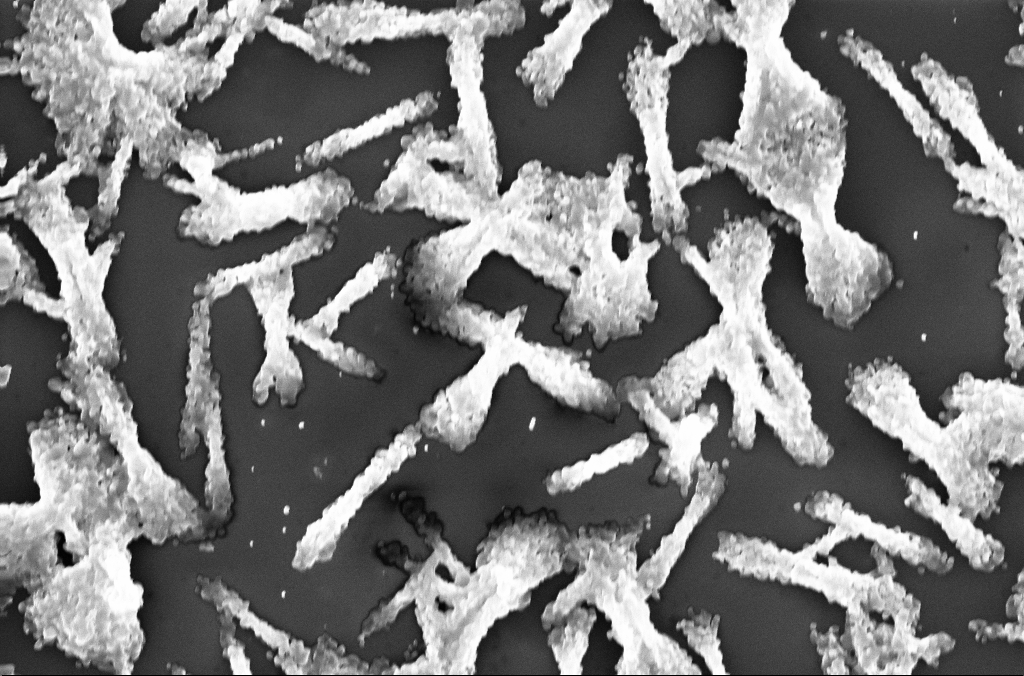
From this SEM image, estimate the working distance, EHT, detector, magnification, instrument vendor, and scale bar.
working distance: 2.8 mm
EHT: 20 kV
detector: InLens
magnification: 10 K X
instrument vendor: Zeiss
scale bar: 2000 nm